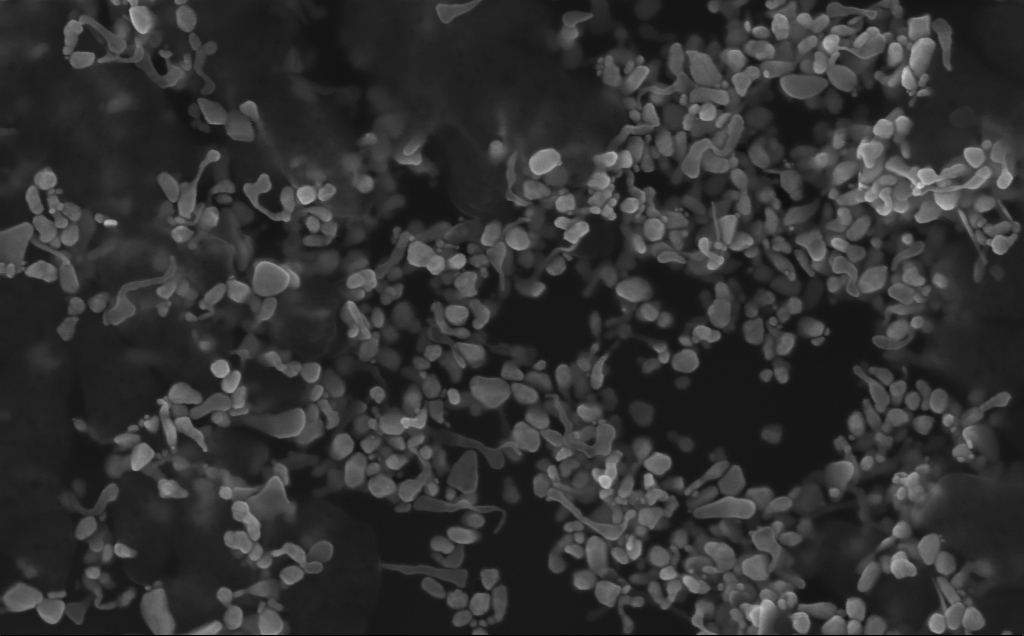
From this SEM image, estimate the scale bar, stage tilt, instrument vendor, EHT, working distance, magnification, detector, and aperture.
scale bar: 200 nm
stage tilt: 0°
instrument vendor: Zeiss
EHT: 10 kV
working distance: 3 mm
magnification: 122.42 K X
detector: InLens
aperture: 30 µm